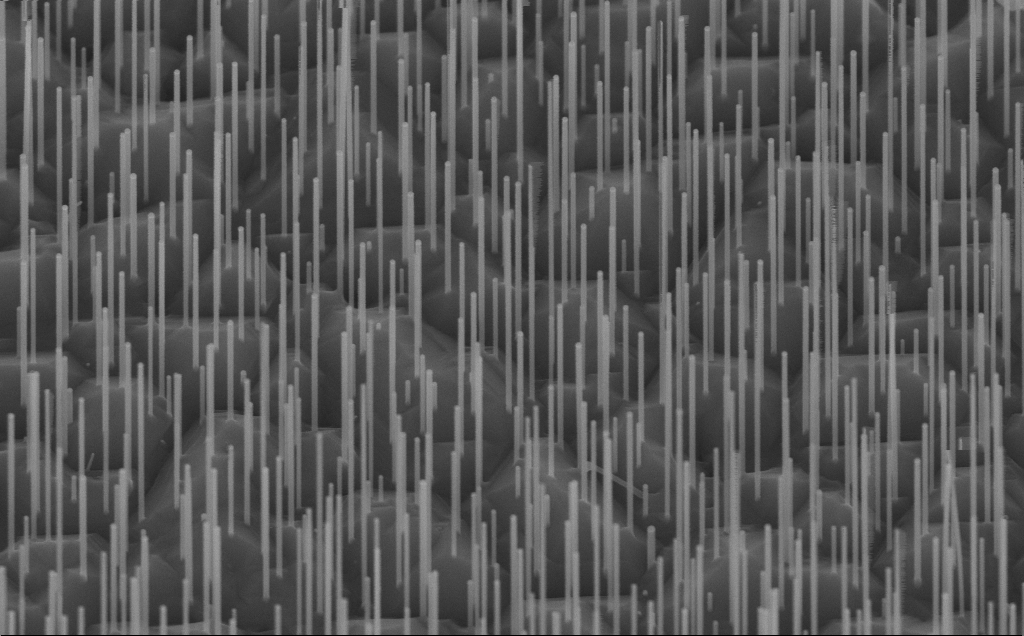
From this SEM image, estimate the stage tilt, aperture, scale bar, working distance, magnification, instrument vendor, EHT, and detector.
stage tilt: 45°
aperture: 30 µm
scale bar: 1000 nm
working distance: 8 mm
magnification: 65.99 K X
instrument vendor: Zeiss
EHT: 10 kV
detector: InLens